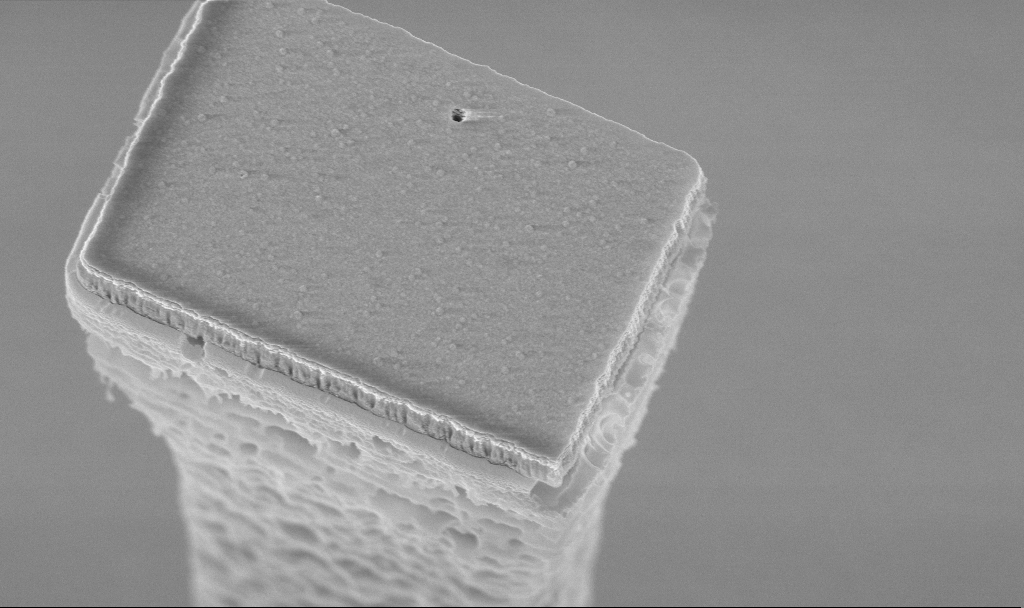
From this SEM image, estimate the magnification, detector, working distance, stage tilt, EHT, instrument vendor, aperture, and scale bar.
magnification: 43.96 K X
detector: InLens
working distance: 4.3 mm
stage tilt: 20°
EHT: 5 kV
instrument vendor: Zeiss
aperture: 30 µm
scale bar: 1000 nm